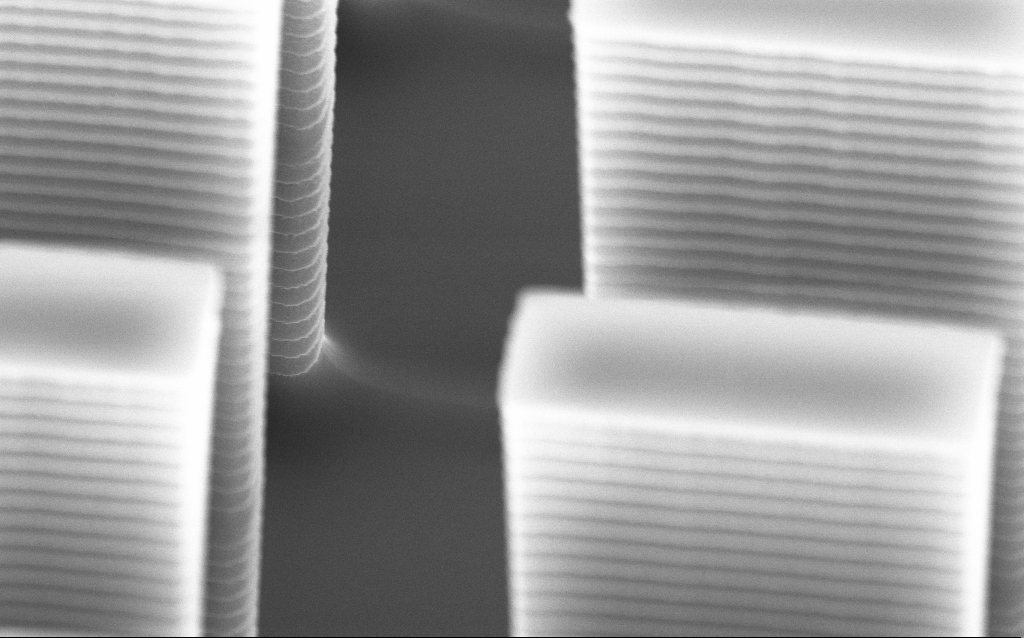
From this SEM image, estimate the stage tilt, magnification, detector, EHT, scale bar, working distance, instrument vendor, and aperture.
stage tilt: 45°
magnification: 36.31 K X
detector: InLens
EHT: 10 kV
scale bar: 1000 nm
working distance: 5.2 mm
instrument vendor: Zeiss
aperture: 30 µm